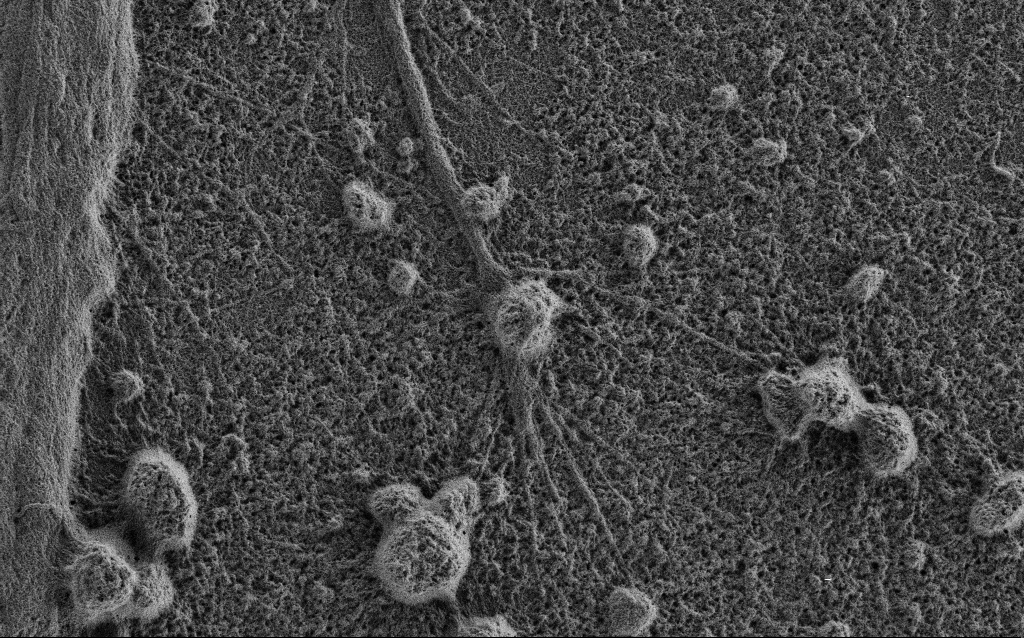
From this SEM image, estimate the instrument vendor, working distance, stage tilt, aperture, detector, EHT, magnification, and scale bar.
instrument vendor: Zeiss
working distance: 3.4 mm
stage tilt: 0°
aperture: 30 µm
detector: SE2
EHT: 0.9 kV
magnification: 10 K X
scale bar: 2000 nm